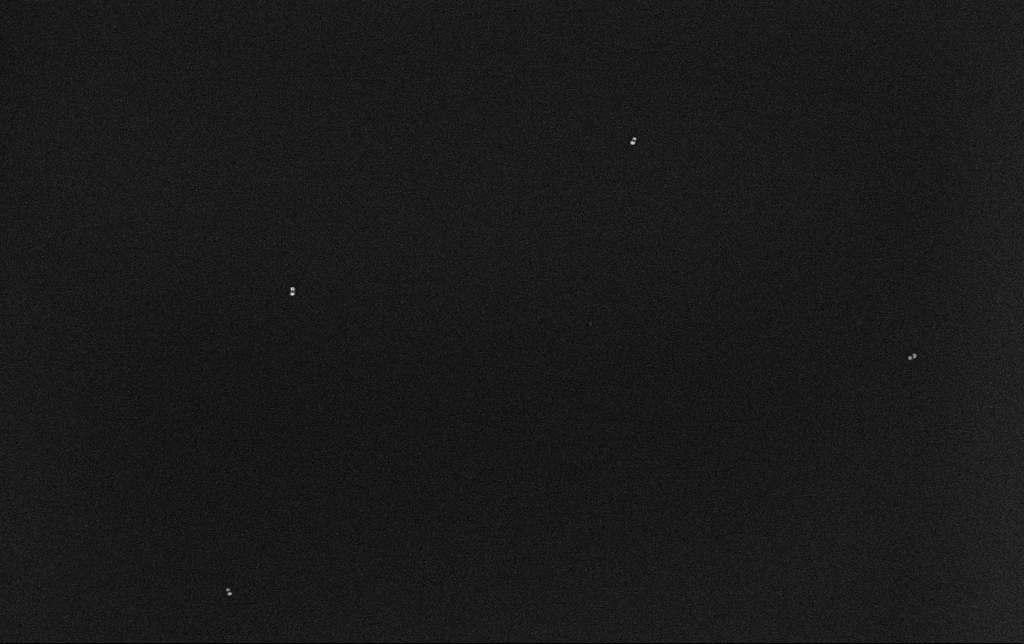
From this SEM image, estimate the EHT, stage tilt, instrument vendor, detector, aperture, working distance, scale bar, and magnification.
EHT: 10 kV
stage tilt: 0°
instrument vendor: Zeiss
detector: InLens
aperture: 30 µm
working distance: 3.2 mm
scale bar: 200 nm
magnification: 100 K X